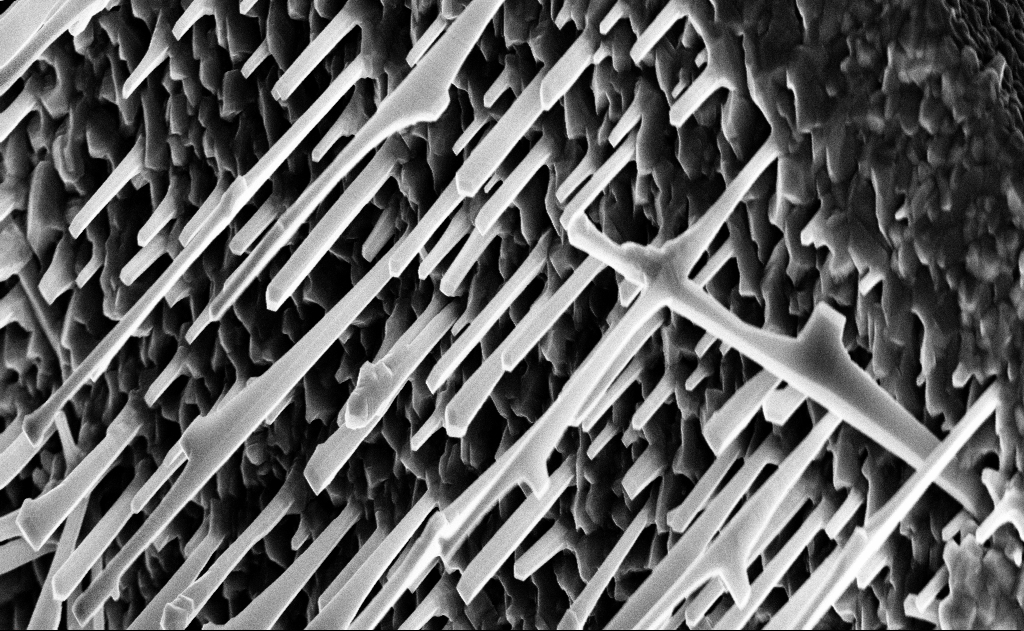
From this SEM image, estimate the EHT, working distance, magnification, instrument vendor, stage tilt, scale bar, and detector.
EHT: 10 kV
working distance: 15 mm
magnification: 20 K X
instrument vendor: Zeiss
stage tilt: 0°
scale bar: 2000 nm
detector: InLens